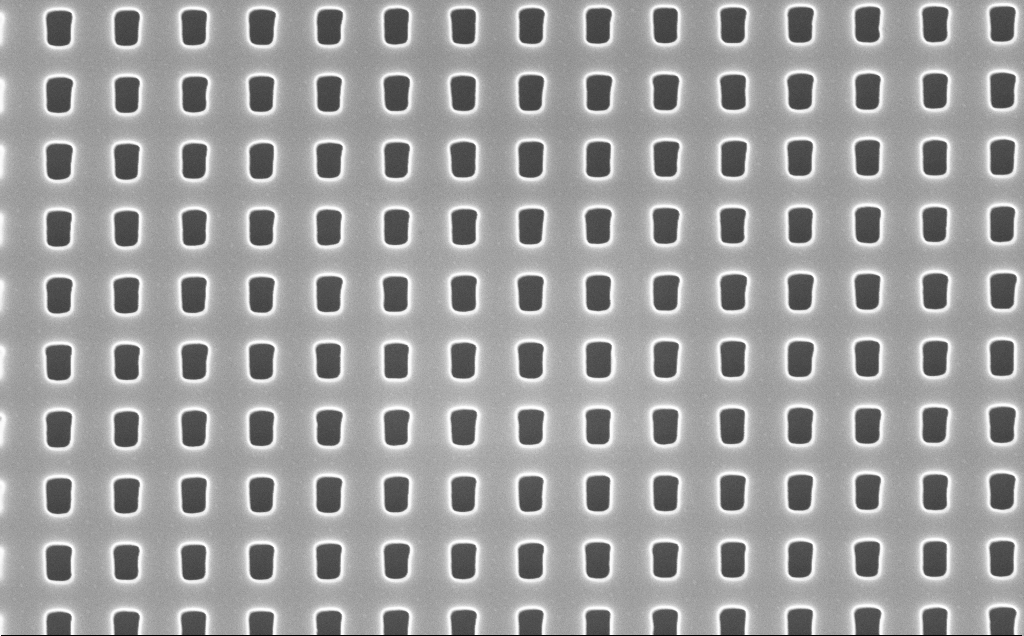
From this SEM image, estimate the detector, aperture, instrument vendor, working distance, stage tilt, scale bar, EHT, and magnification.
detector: InLens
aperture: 30 µm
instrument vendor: Zeiss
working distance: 5 mm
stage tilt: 0°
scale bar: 1000 nm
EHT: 10 kV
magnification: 50 K X